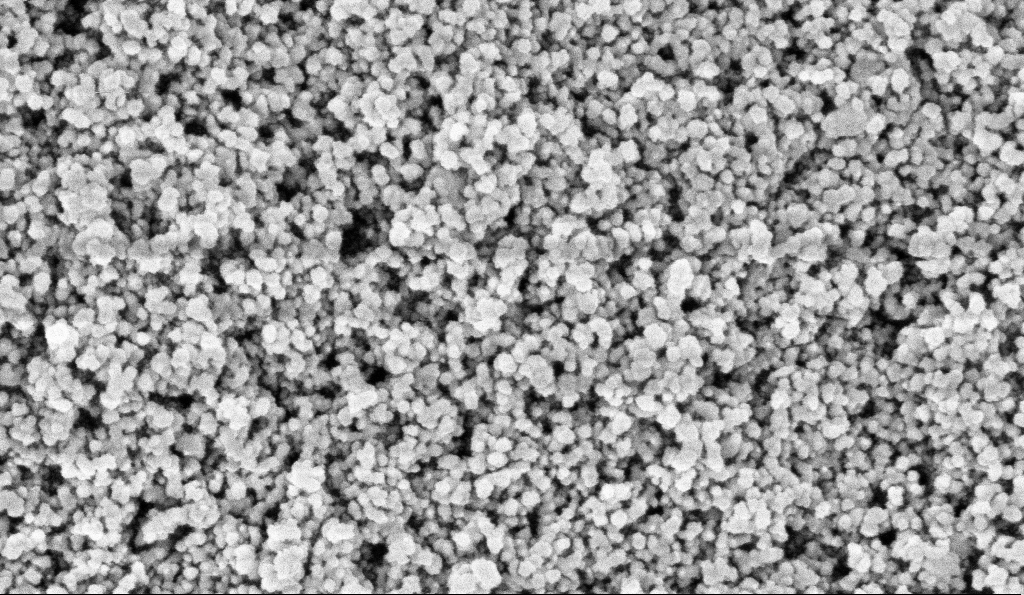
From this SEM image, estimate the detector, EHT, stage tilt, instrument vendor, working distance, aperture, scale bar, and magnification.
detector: InLens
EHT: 5 kV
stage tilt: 0°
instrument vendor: Zeiss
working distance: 6 mm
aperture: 30 µm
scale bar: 100 nm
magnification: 135 K X